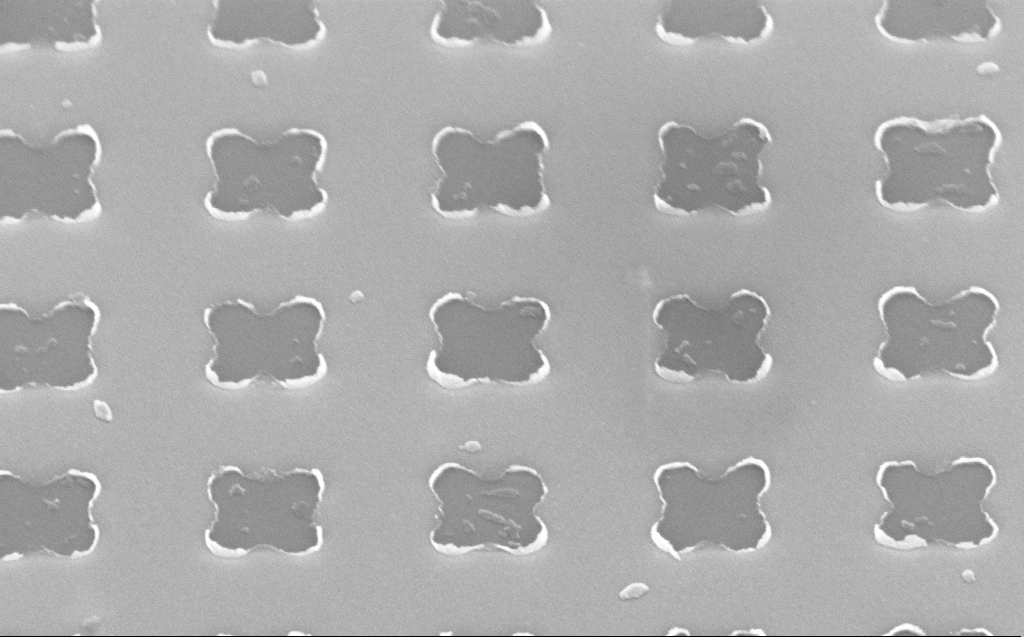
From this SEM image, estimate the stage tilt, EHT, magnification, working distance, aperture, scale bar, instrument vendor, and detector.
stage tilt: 40.6°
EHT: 10 kV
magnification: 166.43 K X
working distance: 5 mm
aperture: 30 µm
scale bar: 200 nm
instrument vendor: Zeiss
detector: InLens